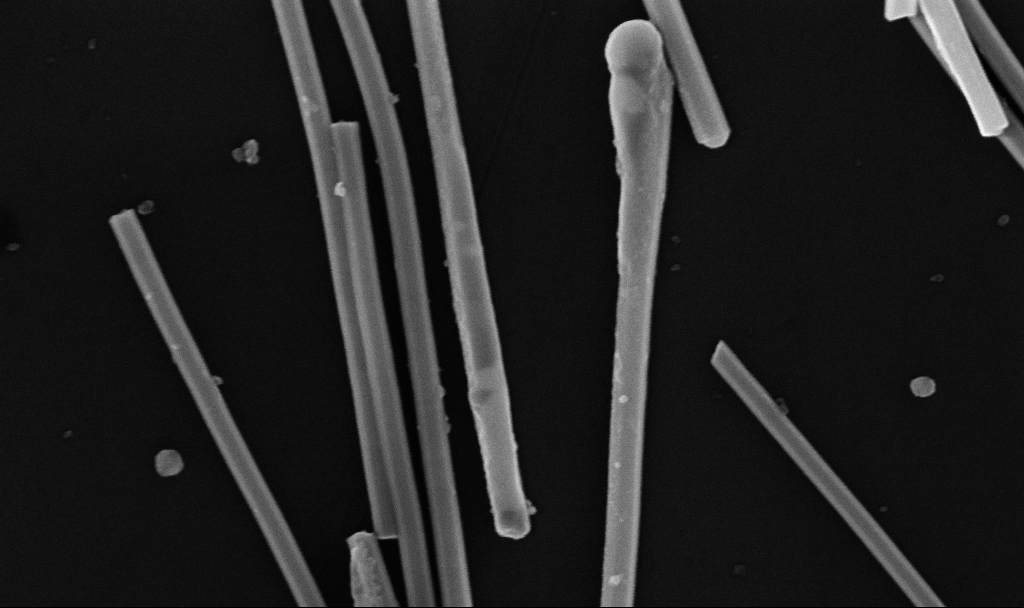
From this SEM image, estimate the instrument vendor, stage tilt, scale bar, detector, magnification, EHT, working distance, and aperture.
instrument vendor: Zeiss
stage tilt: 0°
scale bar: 200 nm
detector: InLens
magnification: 100 K X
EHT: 10 kV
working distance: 6.7 mm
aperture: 30 µm